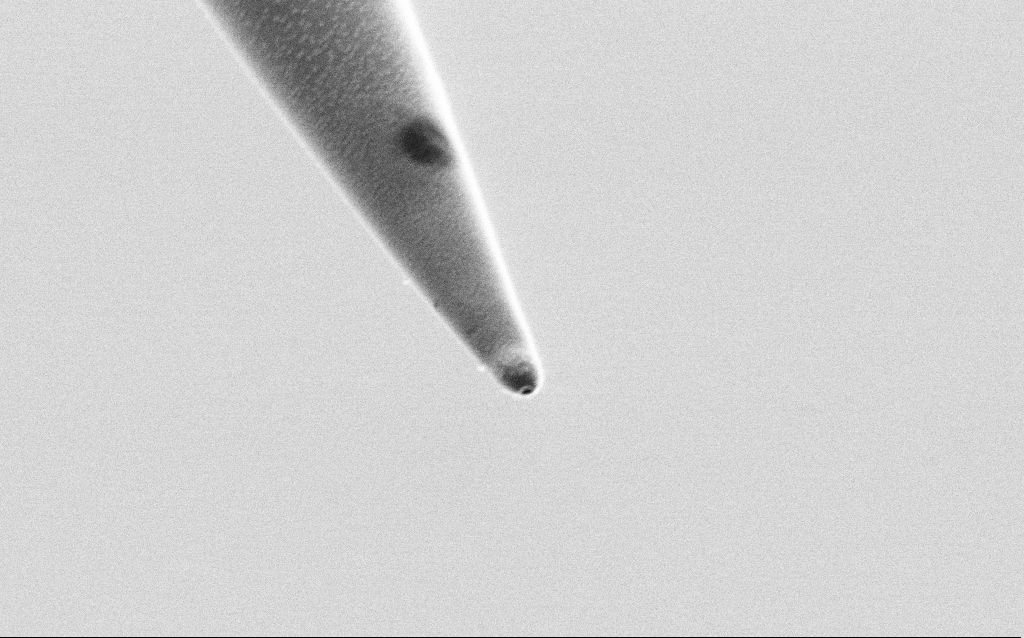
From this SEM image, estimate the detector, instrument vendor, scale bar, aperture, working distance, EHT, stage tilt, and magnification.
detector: SE2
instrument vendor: Zeiss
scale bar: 1000 nm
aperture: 30 µm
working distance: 7.2 mm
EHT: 1 kV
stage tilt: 45°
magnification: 50 K X